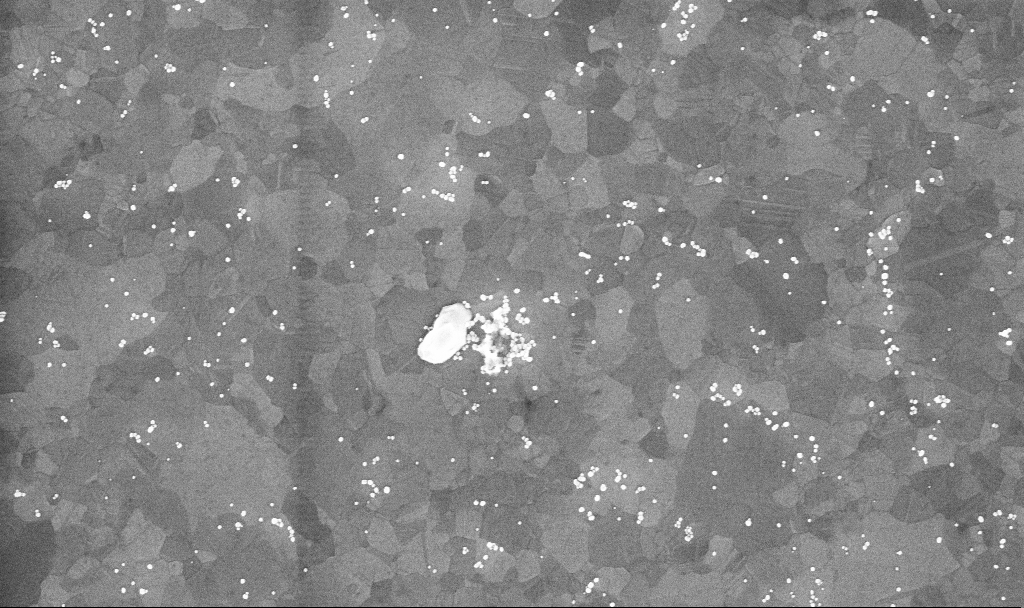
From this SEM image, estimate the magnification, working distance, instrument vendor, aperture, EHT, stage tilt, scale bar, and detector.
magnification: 70.63 K X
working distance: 3.4 mm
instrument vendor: Zeiss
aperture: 30 µm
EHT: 10 kV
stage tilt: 0°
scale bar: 1000 nm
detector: InLens